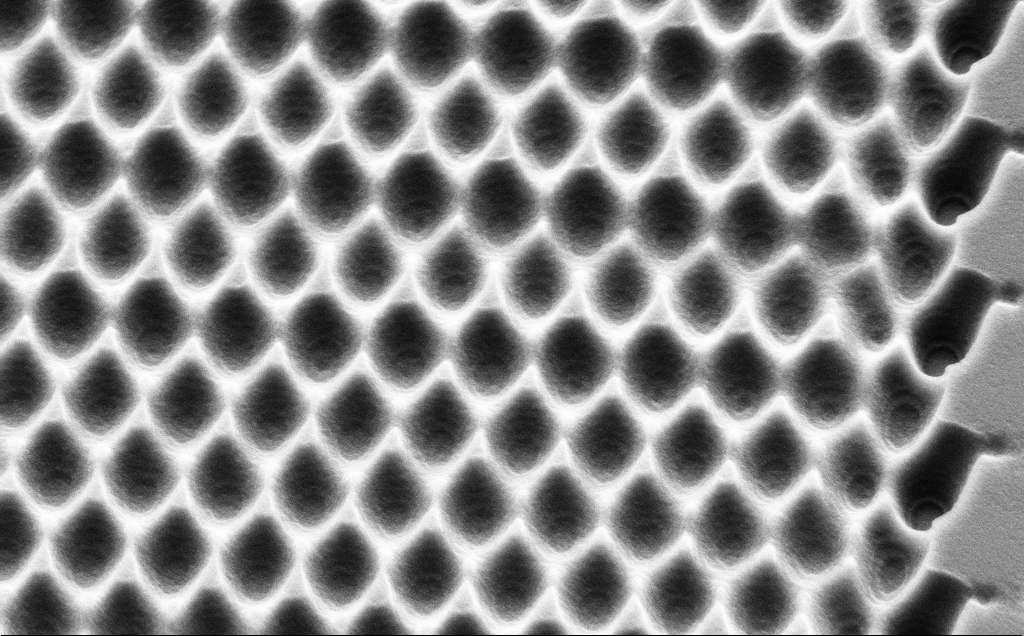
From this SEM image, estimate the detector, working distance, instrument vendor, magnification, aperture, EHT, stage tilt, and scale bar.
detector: SE2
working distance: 10 mm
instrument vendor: Zeiss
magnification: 15.44 K X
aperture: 30 µm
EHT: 5 kV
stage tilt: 45°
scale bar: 2000 nm